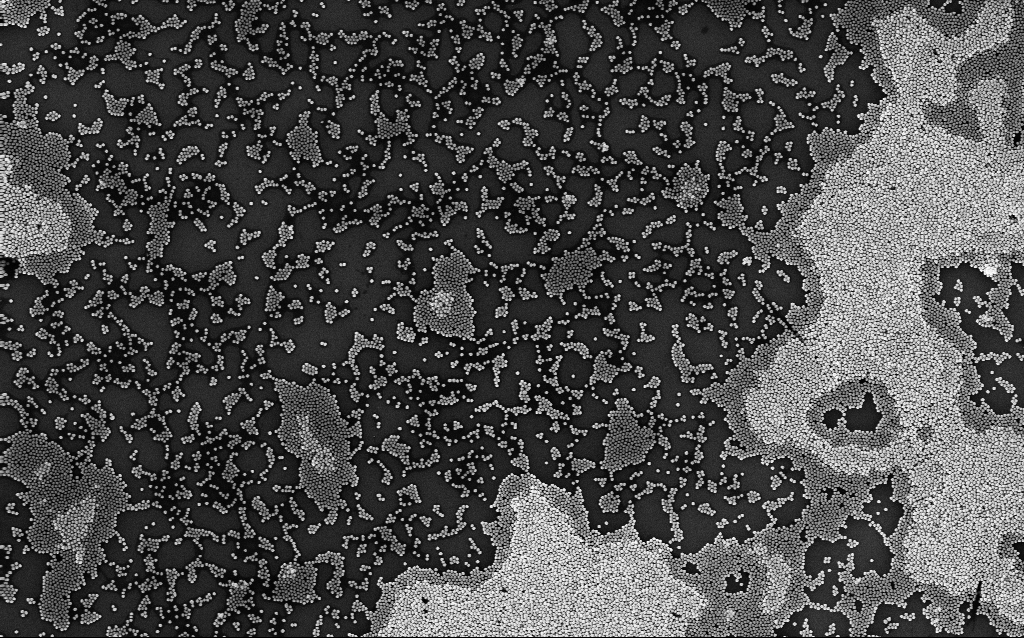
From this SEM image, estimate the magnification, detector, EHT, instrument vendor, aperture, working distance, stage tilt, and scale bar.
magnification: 19.87 K X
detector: InLens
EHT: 8 kV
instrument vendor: Zeiss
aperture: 30 µm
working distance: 3.1 mm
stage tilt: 0°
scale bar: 1000 nm